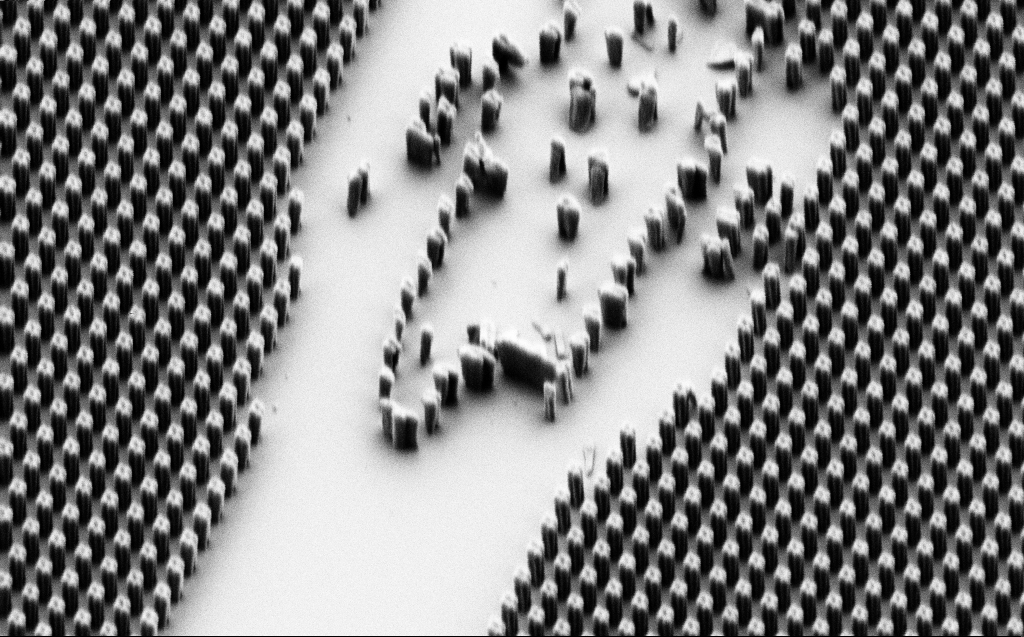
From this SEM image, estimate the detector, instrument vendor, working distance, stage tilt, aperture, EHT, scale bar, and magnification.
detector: SE2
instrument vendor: Zeiss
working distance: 6 mm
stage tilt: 42.8°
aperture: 30 µm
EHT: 1 kV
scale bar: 1000 nm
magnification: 21.51 K X